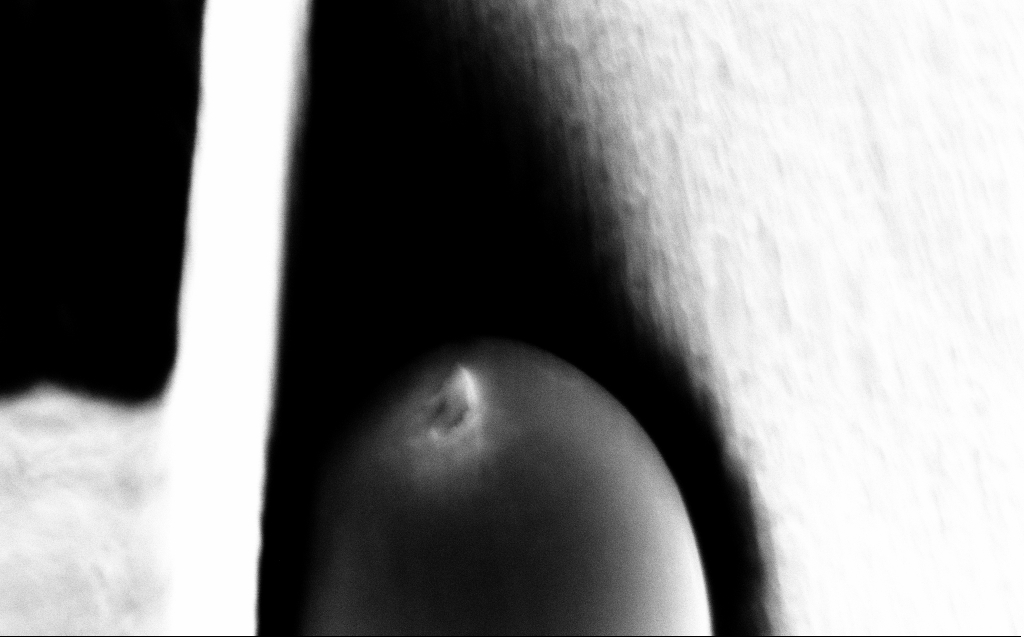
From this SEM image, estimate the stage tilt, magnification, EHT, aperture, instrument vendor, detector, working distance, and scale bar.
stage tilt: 45.1°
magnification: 62.41 K X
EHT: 10 kV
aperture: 120 µm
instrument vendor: Zeiss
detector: InLens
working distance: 7 mm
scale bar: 1000 nm